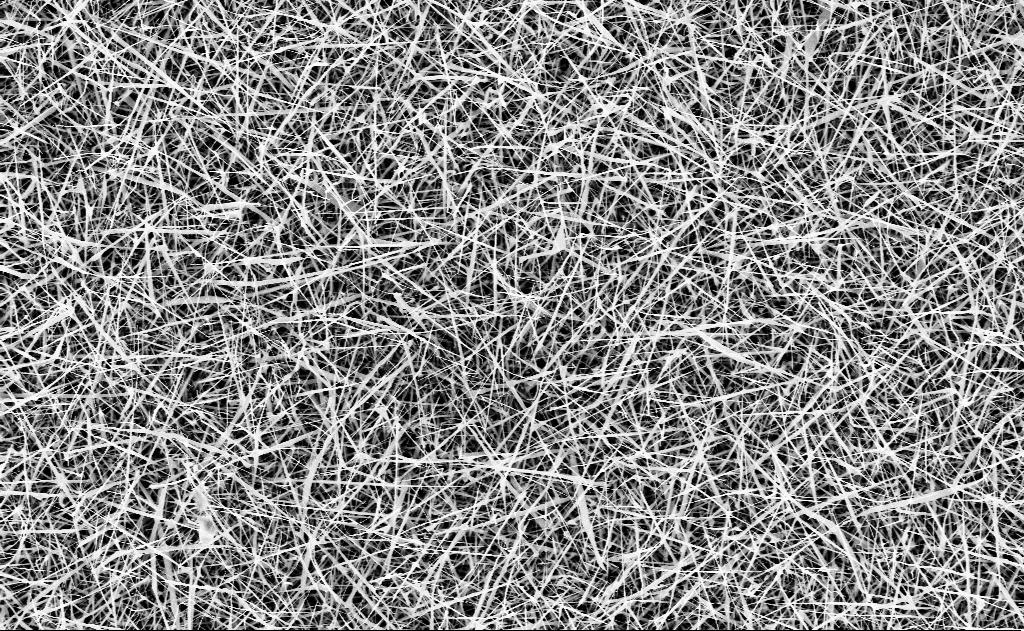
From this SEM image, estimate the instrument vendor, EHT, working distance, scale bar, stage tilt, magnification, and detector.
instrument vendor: Zeiss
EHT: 10 kV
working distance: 15 mm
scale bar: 2000 nm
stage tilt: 0°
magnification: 10 K X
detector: InLens